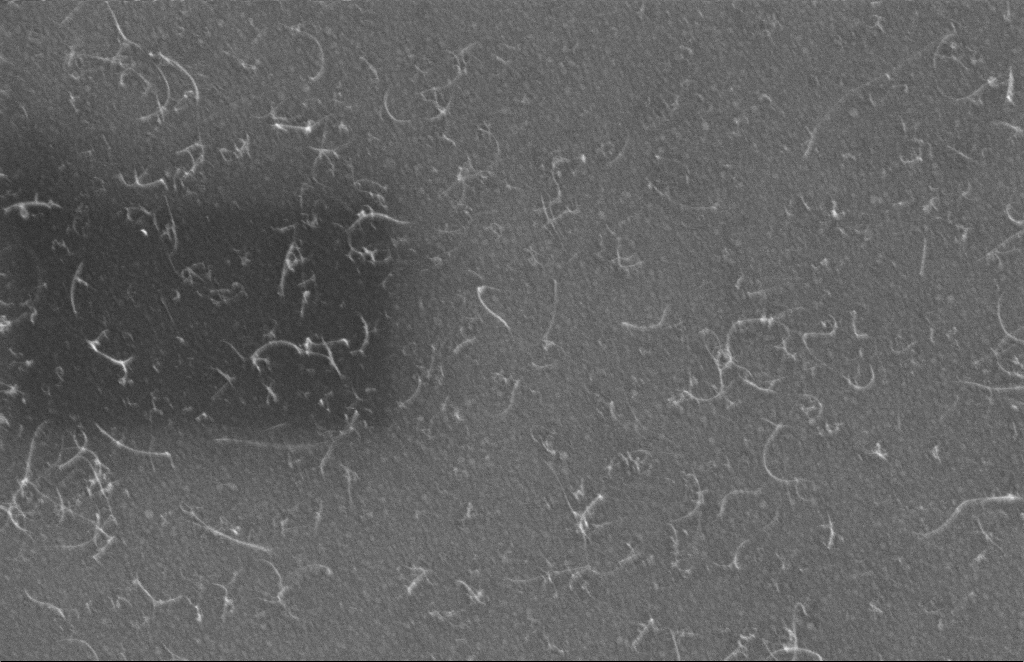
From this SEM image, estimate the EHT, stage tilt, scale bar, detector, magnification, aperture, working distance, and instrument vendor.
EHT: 5 kV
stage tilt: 0°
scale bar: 200 nm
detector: InLens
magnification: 121.85 K X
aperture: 20 µm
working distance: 4 mm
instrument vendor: Zeiss